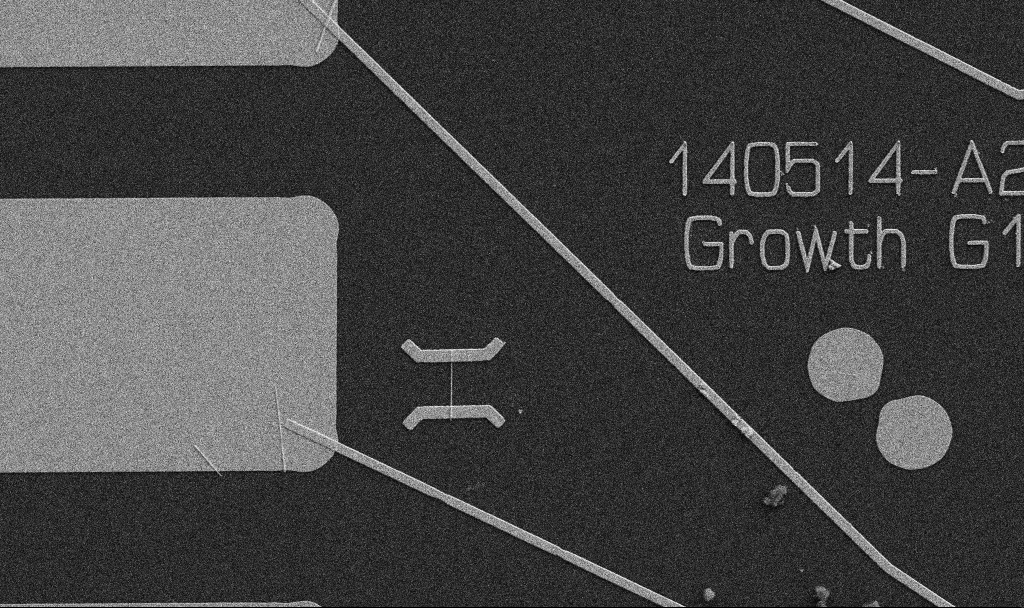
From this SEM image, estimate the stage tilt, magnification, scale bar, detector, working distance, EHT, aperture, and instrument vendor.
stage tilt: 0°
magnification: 5 K X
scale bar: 10000 nm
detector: SE2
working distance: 10.7 mm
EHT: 5 kV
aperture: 30 µm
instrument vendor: Zeiss